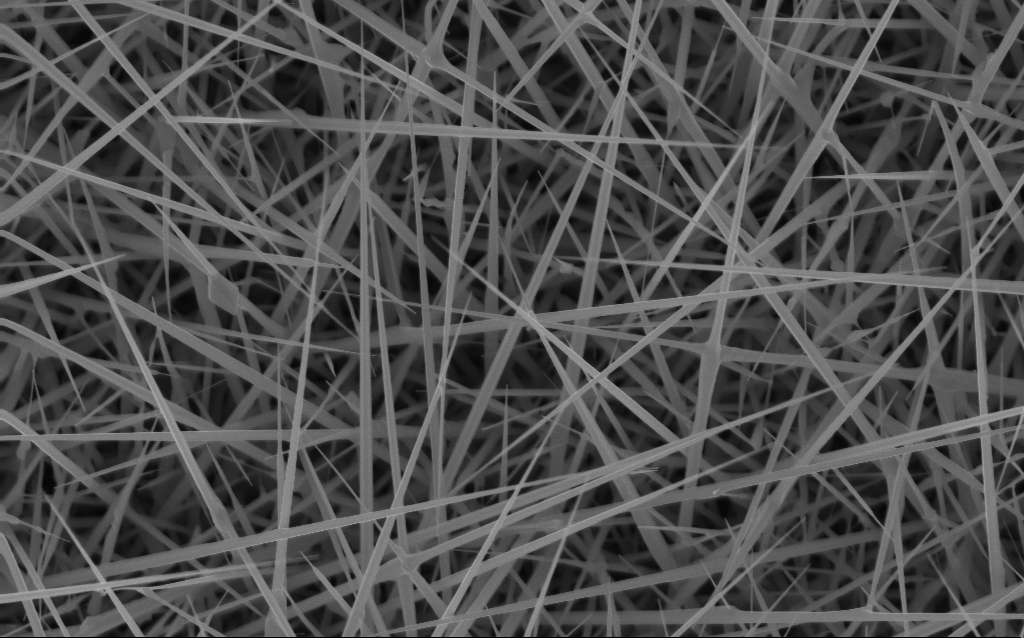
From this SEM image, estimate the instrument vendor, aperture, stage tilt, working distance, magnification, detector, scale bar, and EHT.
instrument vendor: Zeiss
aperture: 30 µm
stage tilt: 0°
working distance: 4 mm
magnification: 20 K X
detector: InLens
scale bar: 2000 nm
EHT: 10 kV